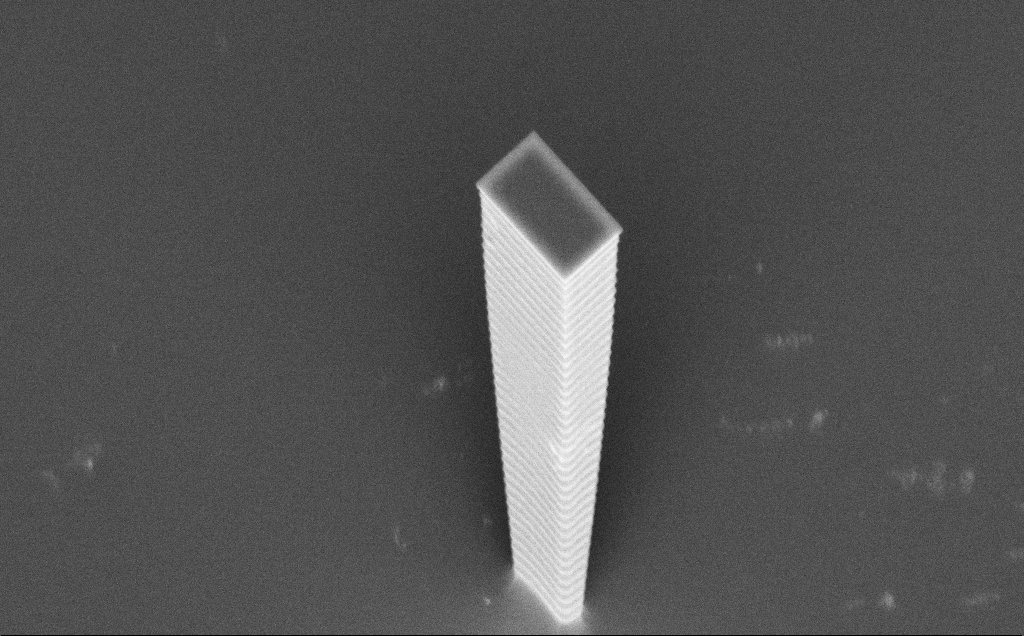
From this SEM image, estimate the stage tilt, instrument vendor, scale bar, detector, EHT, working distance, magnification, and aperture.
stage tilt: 45°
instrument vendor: Zeiss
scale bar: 2000 nm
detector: InLens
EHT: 7.5 kV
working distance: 8 mm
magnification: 10.19 K X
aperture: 30 µm